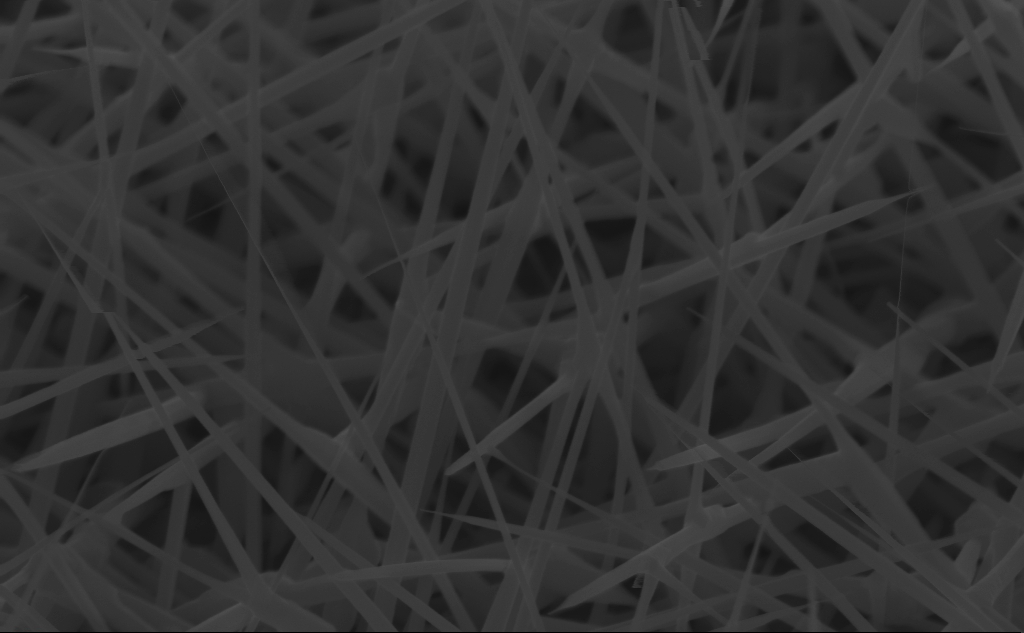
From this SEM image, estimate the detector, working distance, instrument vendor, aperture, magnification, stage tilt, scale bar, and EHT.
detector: InLens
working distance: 5 mm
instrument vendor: Zeiss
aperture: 30 µm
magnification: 80 K X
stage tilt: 45°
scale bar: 200 nm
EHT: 10 kV